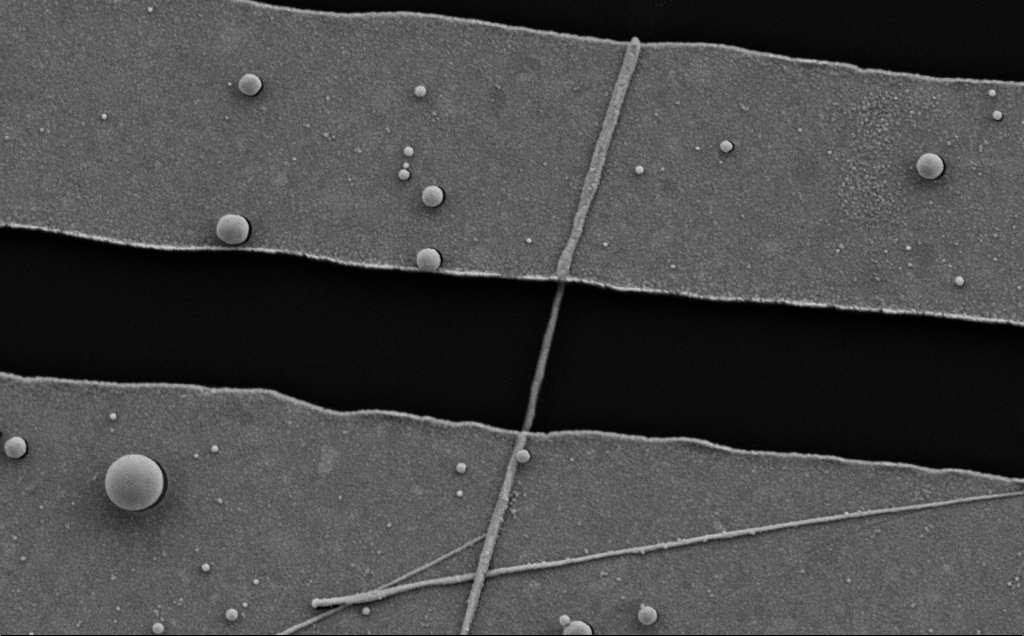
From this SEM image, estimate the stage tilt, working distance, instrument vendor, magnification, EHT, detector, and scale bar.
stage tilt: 0°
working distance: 8 mm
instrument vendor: Zeiss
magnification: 35.53 K X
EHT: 5 kV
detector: SE2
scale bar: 1000 nm